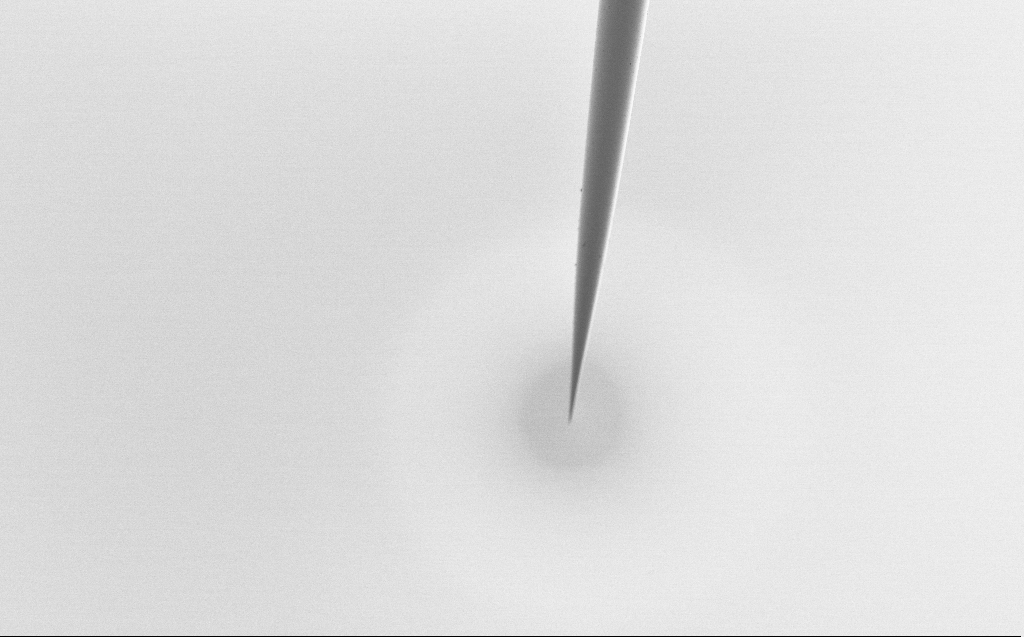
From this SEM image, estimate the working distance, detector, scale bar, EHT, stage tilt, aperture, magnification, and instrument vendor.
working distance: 5 mm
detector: SE2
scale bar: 20000 nm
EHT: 1 kV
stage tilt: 45°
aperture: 30 µm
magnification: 1 K X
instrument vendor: Zeiss